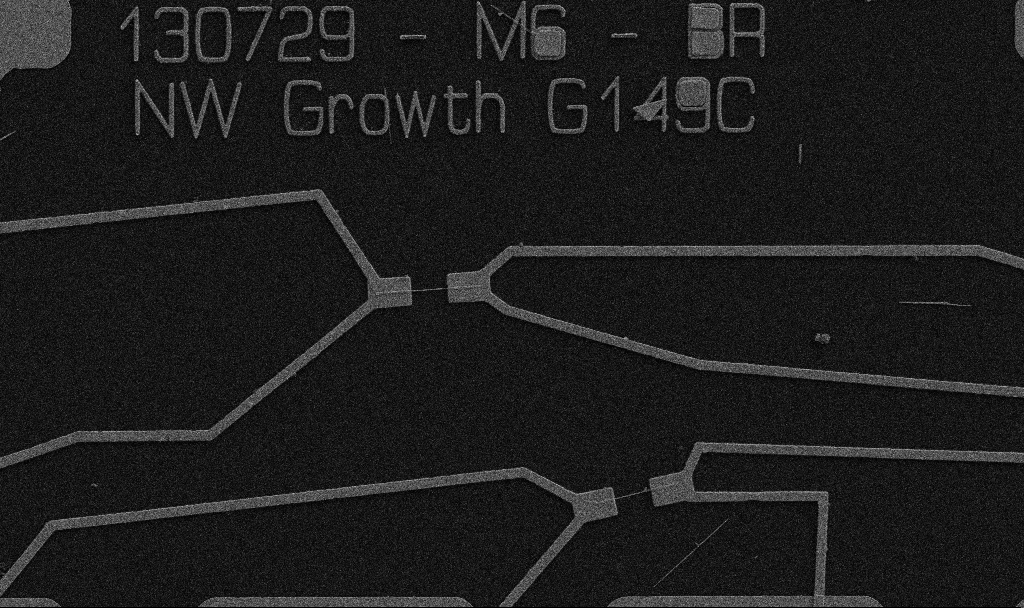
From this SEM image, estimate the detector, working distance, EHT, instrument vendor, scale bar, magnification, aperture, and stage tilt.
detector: SE2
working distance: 10.7 mm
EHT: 5 kV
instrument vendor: Zeiss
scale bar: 10000 nm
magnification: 5 K X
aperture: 30 µm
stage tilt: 0°